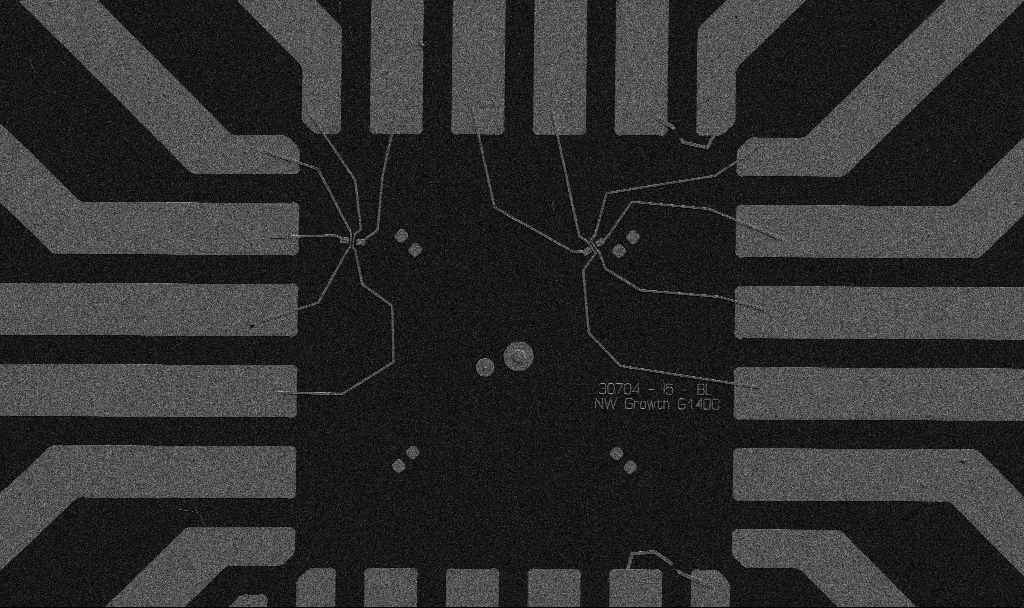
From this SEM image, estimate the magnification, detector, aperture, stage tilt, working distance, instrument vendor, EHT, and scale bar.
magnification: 1 K X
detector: SE2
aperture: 30 µm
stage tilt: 0°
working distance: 10.7 mm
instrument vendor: Zeiss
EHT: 5 kV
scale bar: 20000 nm